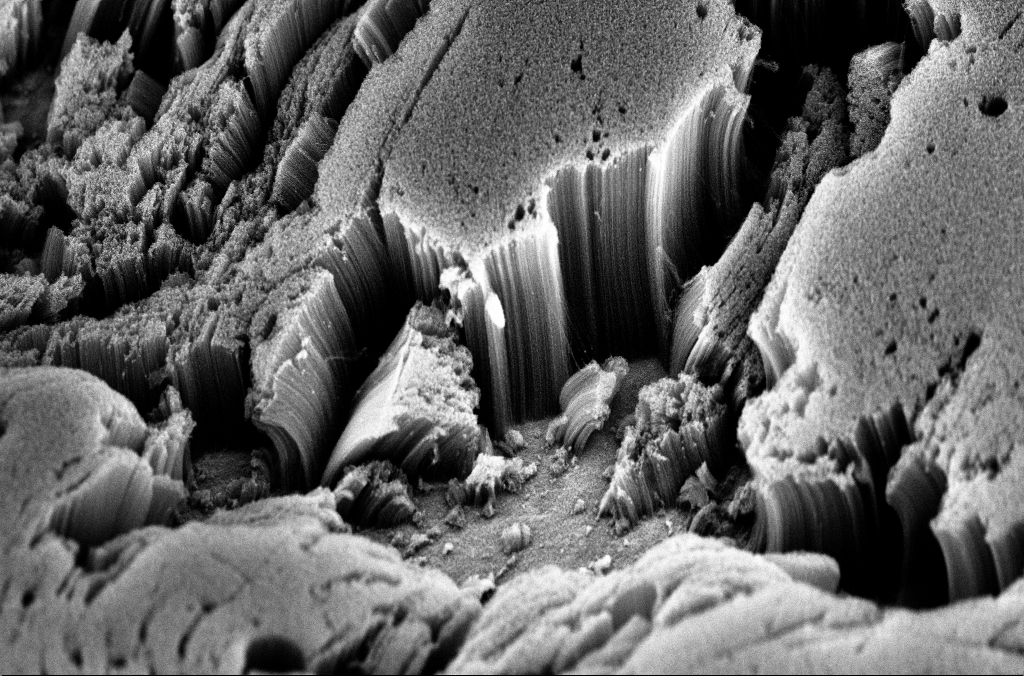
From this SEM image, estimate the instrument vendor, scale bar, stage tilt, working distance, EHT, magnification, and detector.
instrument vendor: Zeiss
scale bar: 20000 nm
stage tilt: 45°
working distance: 3.4 mm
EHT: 3 kV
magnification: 1 K X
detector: InLens